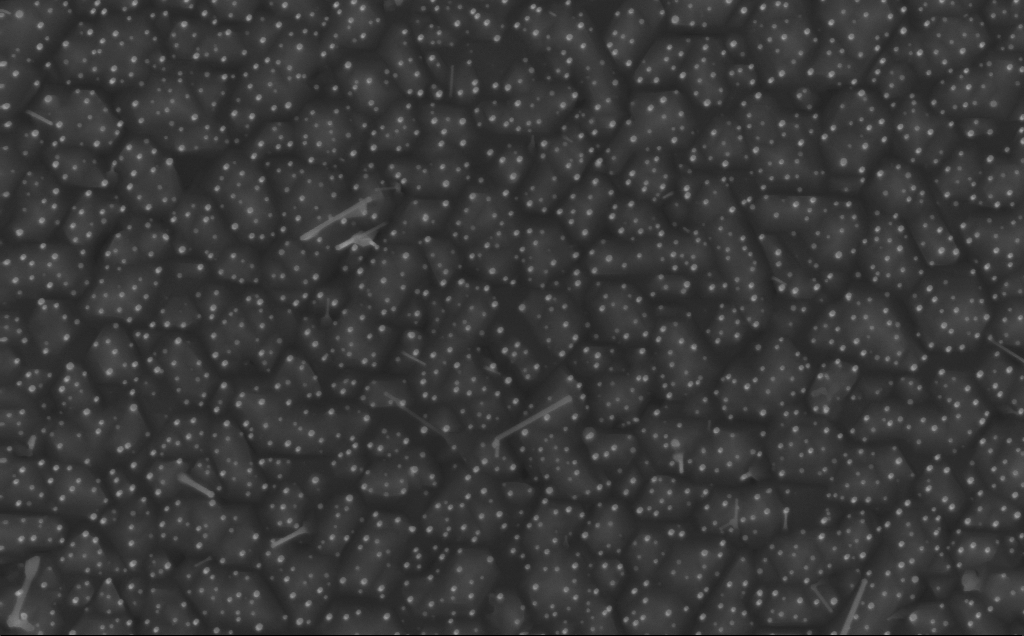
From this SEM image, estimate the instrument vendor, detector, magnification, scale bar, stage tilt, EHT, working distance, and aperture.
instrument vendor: Zeiss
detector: InLens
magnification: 40 K X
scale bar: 1000 nm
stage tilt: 0°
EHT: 10 kV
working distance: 5 mm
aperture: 30 µm